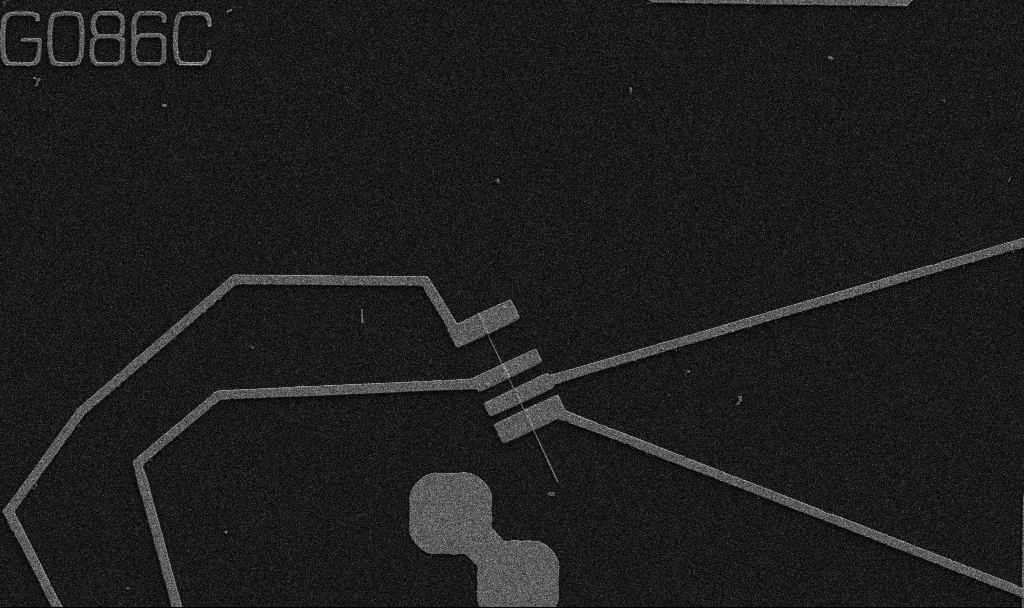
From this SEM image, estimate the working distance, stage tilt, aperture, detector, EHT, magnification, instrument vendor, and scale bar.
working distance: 10.7 mm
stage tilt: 0°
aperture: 30 µm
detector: SE2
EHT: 5 kV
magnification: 5 K X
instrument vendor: Zeiss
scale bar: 10000 nm